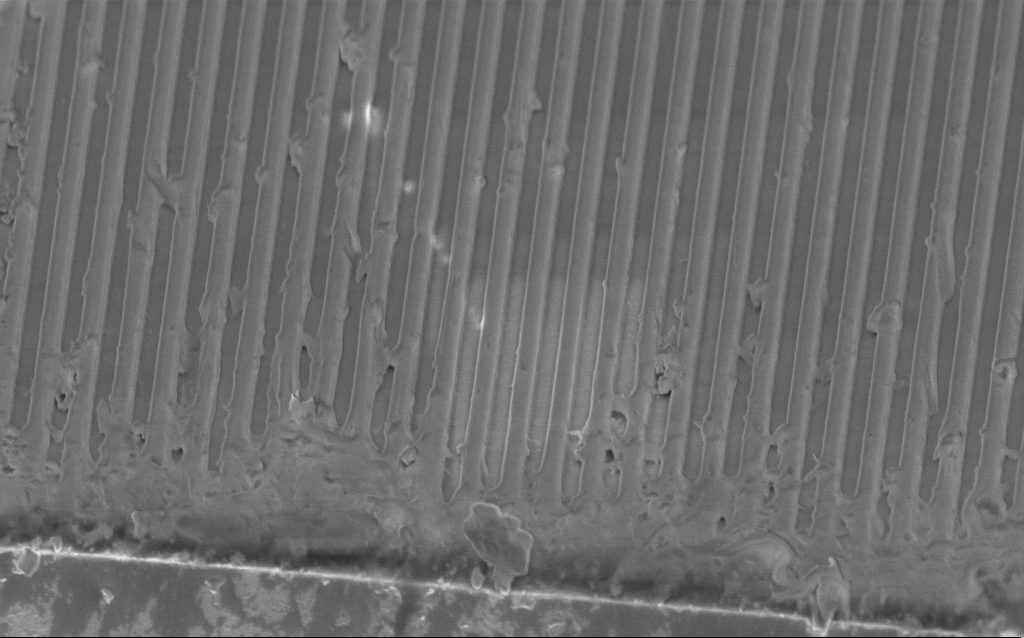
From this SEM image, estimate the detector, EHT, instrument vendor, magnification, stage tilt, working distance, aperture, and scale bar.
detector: InLens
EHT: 2 kV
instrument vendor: Zeiss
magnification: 29.27 K X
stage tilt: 45°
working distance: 3.6 mm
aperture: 30 µm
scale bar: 1000 nm